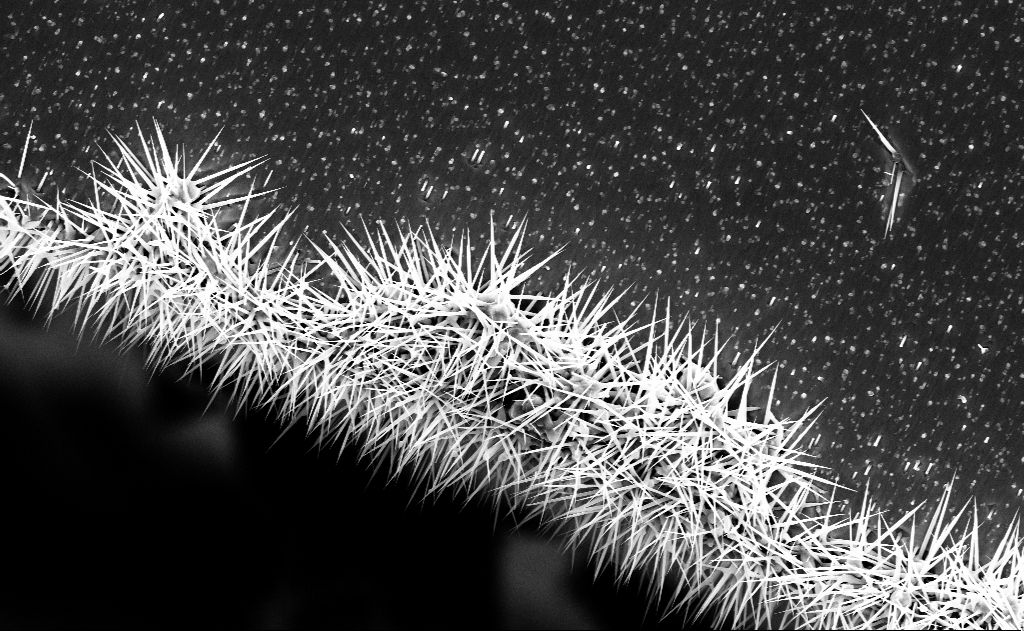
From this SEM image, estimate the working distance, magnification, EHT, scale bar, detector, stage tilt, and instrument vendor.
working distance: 9 mm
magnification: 10 K X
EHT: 10 kV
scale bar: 2000 nm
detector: InLens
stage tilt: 0°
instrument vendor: Zeiss